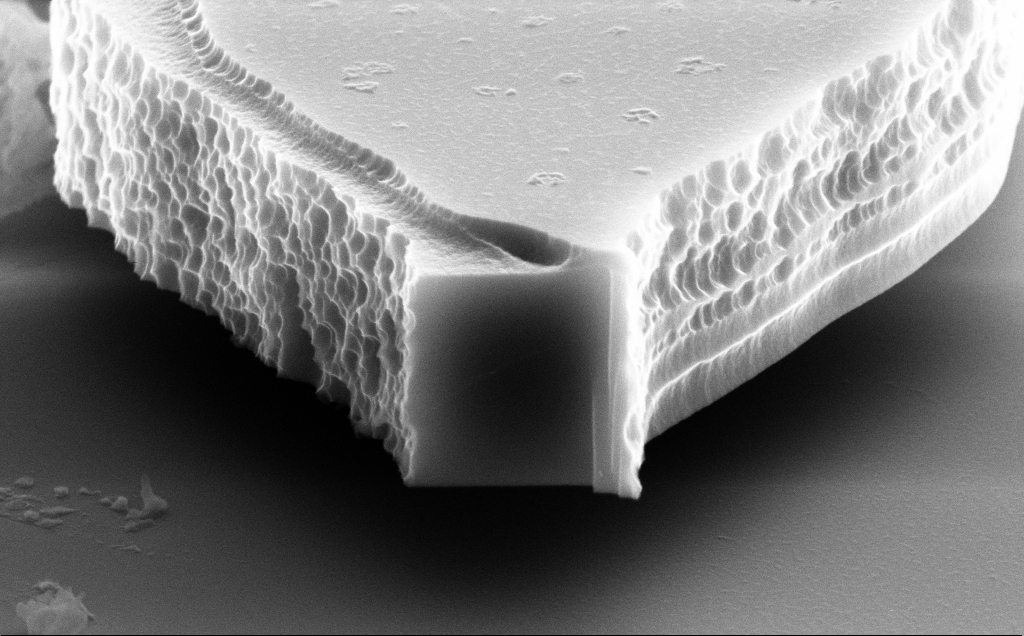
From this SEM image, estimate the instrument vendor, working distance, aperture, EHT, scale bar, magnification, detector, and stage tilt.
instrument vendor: Zeiss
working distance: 10 mm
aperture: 30 µm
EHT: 10 kV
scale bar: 1000 nm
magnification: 37.56 K X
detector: SE2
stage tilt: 70°